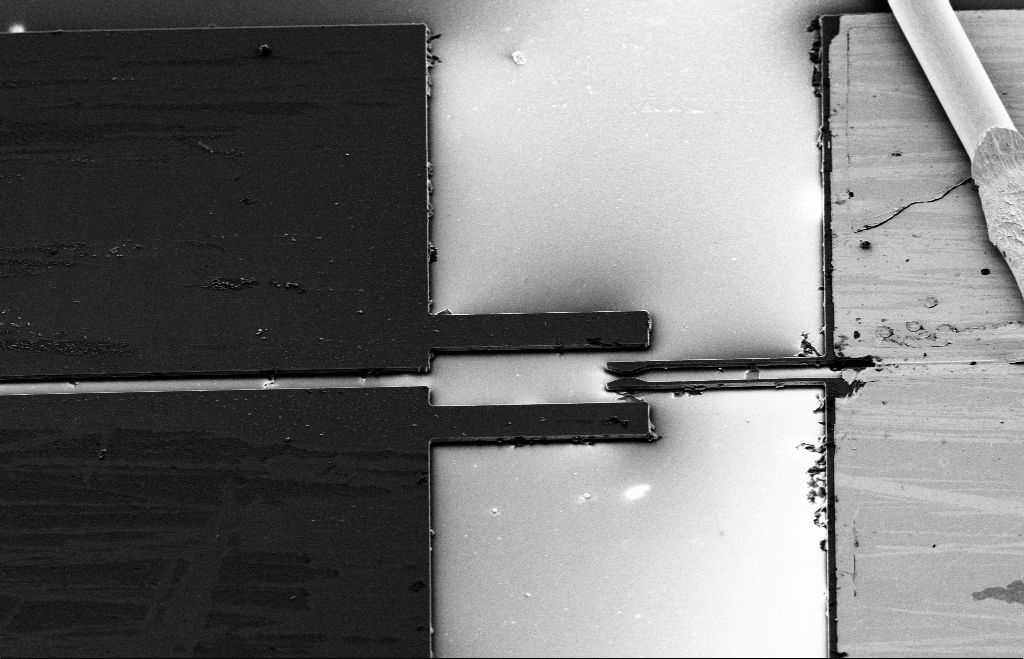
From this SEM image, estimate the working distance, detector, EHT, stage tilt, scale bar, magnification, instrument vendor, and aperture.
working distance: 13 mm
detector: SE2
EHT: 5 kV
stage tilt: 40°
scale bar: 20000 nm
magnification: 0.807 K X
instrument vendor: Zeiss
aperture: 30 µm